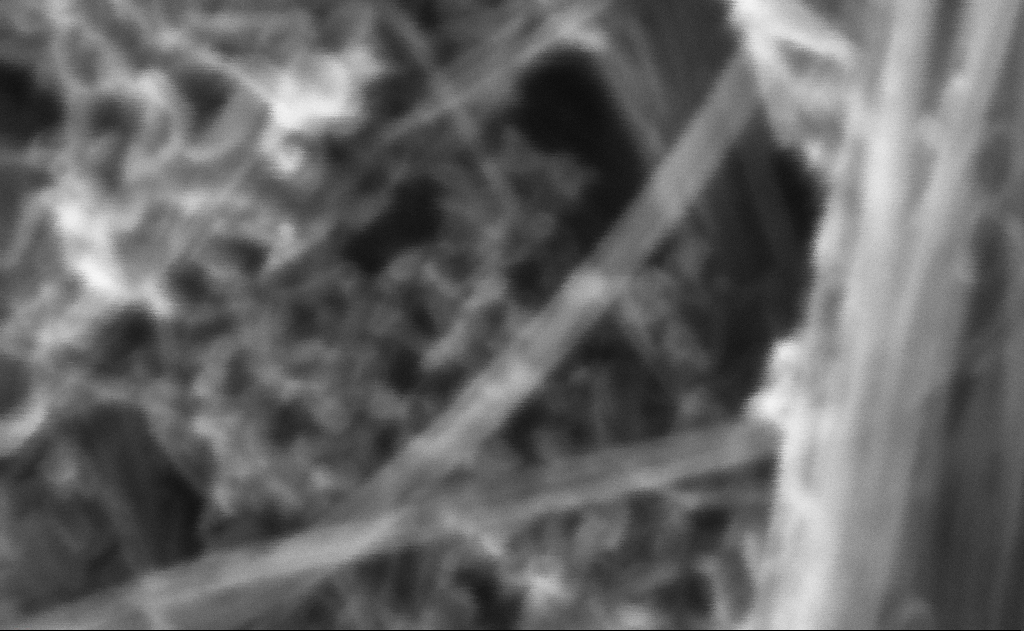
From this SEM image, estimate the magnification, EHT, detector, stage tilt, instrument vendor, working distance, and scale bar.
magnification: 1609.42 K X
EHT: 10 kV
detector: InLens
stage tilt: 0°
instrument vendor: Zeiss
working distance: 3 mm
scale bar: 20 nm